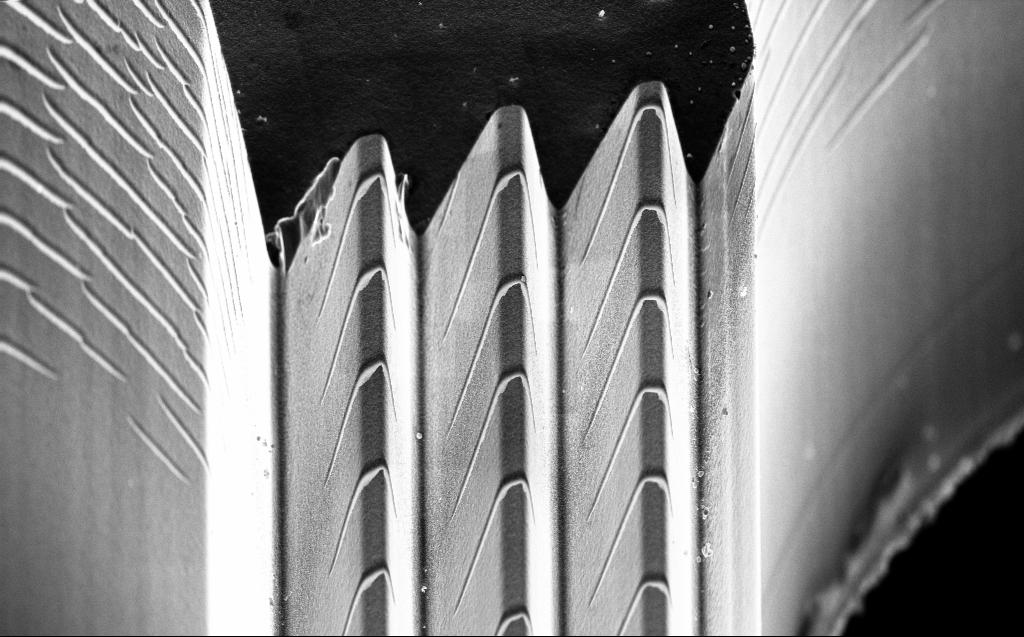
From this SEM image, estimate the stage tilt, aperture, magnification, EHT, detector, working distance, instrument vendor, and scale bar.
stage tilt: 45°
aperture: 30 µm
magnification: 4.66 K X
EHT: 10 kV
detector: InLens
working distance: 4 mm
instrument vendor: Zeiss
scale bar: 10000 nm